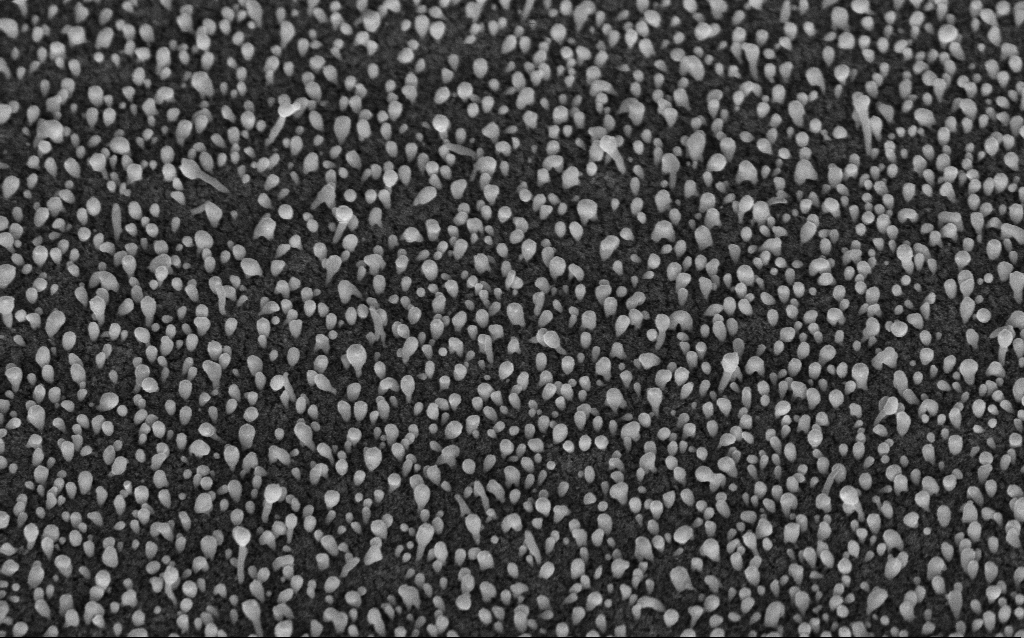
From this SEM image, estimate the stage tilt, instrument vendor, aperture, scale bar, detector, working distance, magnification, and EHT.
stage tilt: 45°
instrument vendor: Zeiss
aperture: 30 µm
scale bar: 1000 nm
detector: InLens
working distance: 6.2 mm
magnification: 50 K X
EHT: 5 kV